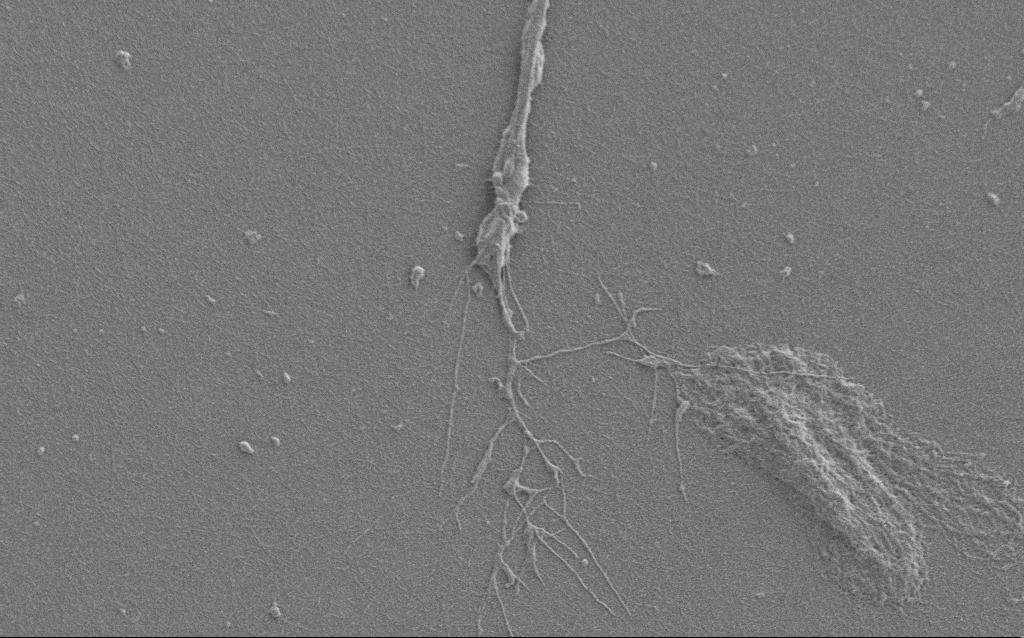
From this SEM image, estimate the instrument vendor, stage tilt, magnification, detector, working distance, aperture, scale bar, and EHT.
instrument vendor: Zeiss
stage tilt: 0°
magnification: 6 K X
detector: SE2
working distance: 6 mm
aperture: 30 µm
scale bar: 10000 nm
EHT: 1 kV